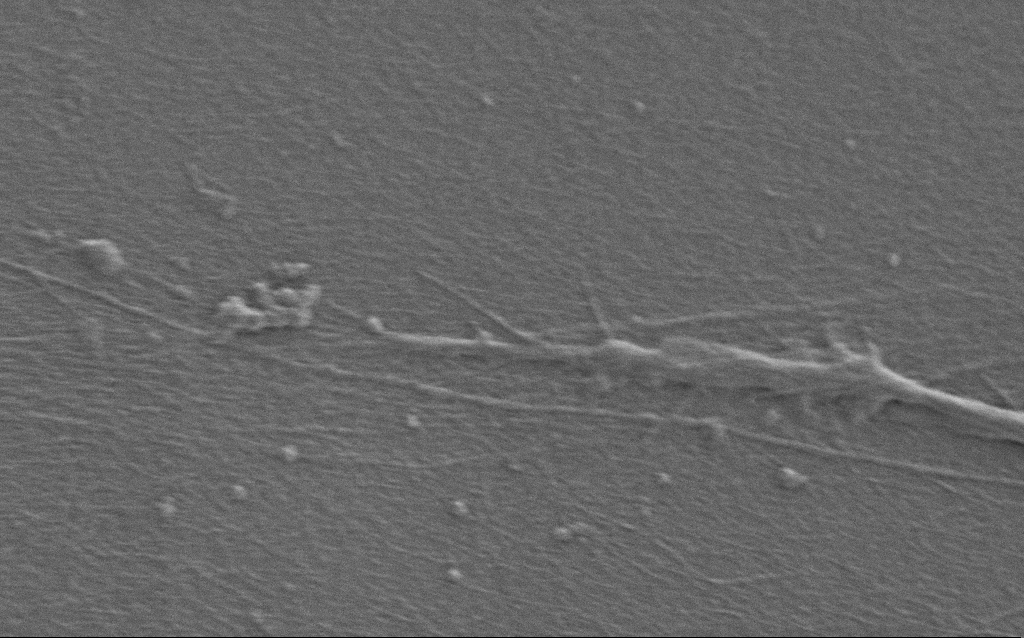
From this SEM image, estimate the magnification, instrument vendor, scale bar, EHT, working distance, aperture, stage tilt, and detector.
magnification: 10 K X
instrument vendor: Zeiss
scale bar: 2000 nm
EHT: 0.9 kV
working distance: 6 mm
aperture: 30 µm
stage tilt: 0°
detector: SE2